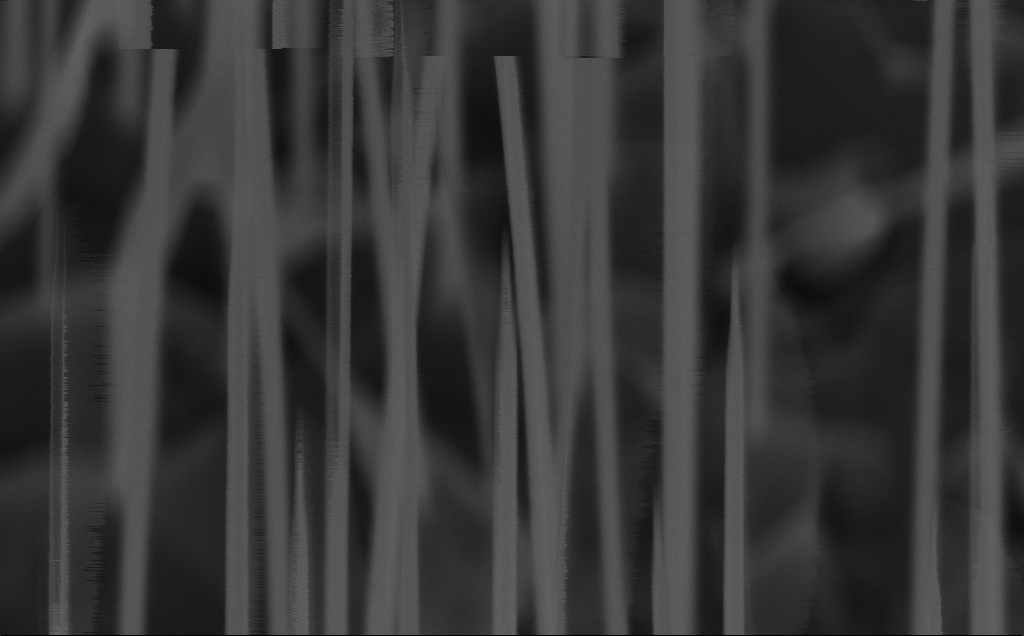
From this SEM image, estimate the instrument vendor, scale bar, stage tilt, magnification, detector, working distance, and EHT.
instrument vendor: Zeiss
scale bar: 200 nm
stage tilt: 45°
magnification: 142.21 K X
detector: InLens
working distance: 8 mm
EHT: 5 kV